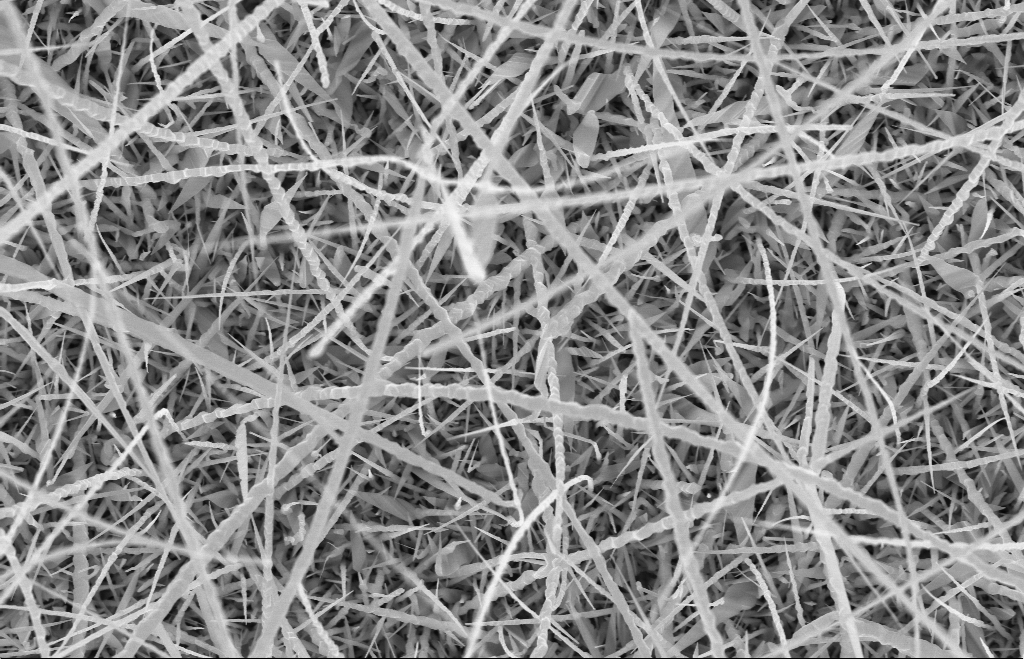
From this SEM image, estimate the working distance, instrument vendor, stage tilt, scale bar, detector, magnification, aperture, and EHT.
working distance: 9 mm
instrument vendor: Zeiss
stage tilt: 0°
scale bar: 1000 nm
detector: InLens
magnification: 20 K X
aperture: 30 µm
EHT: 10 kV